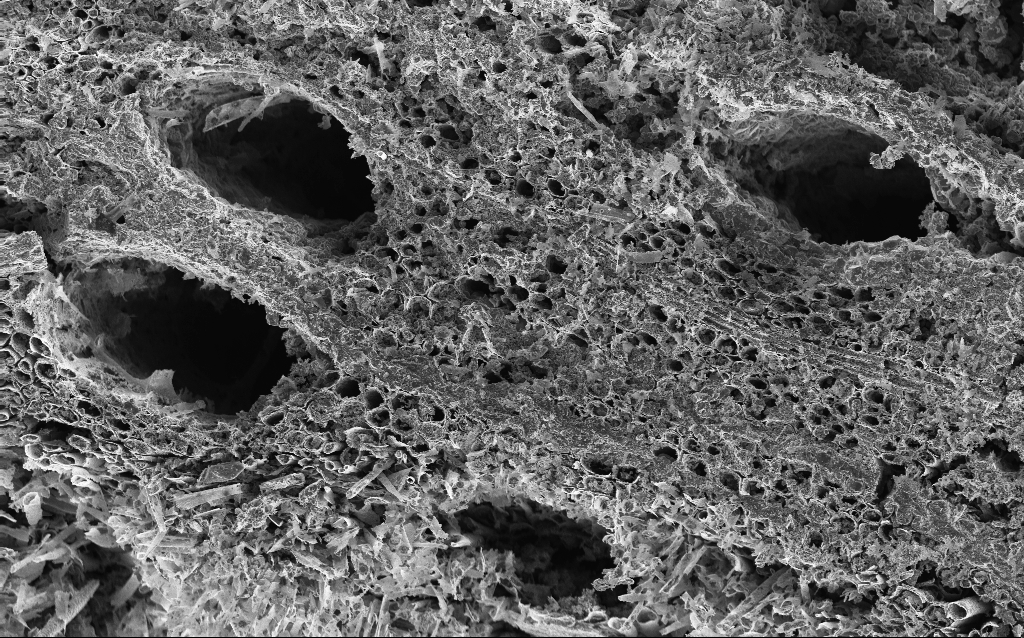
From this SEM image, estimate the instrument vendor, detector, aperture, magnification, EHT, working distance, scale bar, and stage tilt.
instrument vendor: Zeiss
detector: InLens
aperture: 30 µm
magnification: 0.5 K X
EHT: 10 kV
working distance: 3 mm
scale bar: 100000 nm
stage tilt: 0°